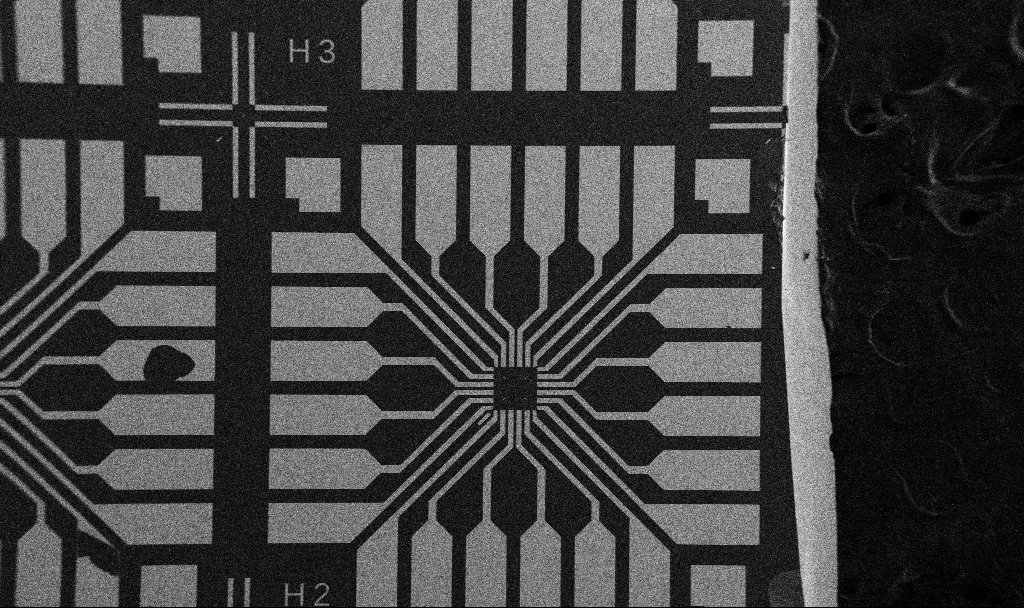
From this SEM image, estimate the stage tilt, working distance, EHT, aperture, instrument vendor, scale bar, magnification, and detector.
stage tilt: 0°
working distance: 10.7 mm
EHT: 5 kV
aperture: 30 µm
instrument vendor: Zeiss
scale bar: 200000 nm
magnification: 0.1 K X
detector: SE2